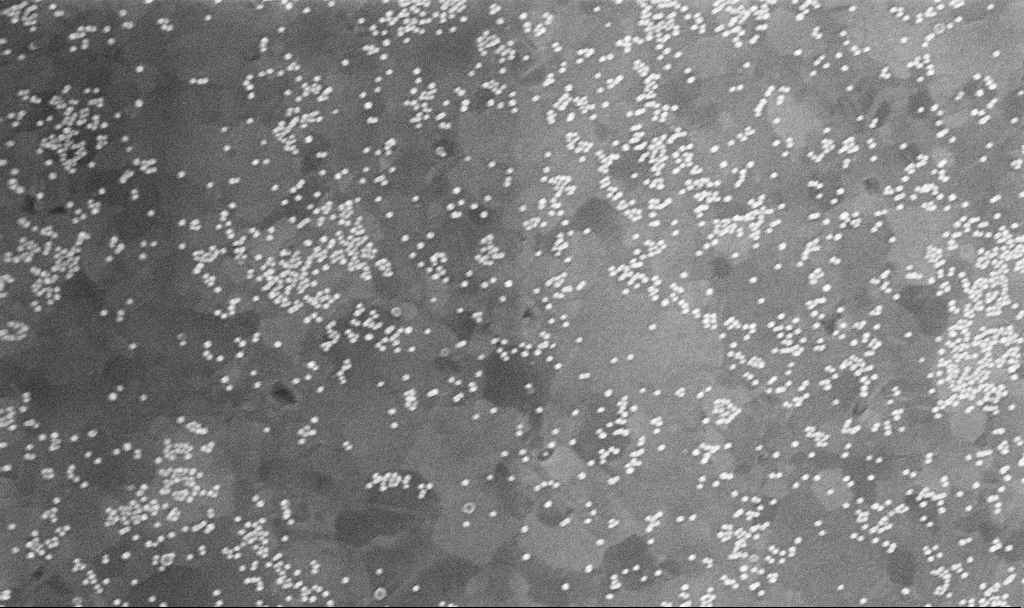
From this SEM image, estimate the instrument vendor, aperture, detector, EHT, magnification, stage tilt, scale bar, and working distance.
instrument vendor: Zeiss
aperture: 30 µm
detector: InLens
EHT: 10 kV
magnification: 100 K X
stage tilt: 0°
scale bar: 200 nm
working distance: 3.7 mm